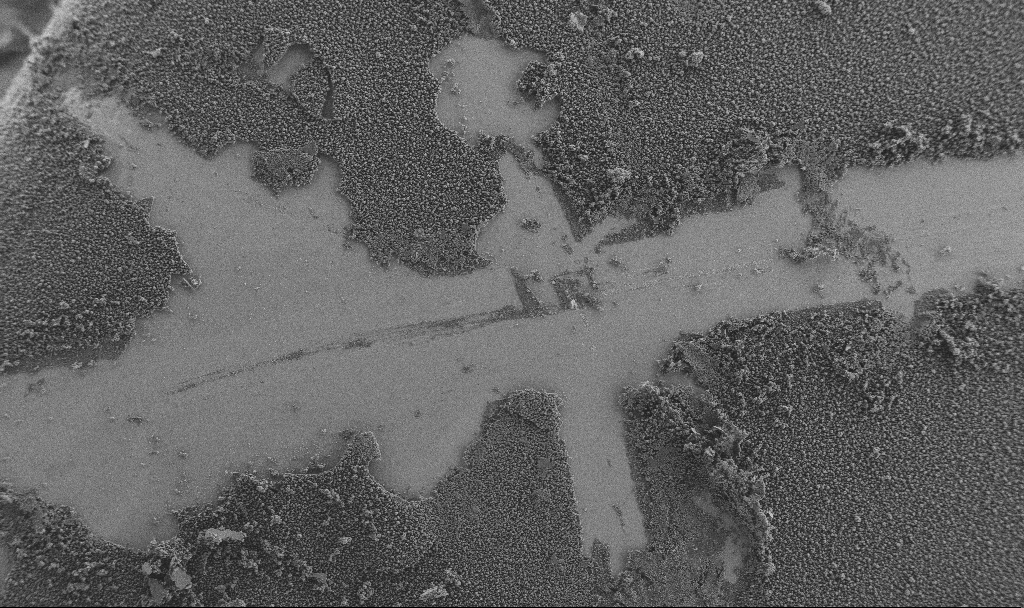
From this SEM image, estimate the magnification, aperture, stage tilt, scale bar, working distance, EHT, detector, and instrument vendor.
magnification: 0.15 K X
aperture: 30 µm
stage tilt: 0°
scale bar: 100000 nm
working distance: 4 mm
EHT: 3 kV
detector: SE2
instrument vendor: Zeiss